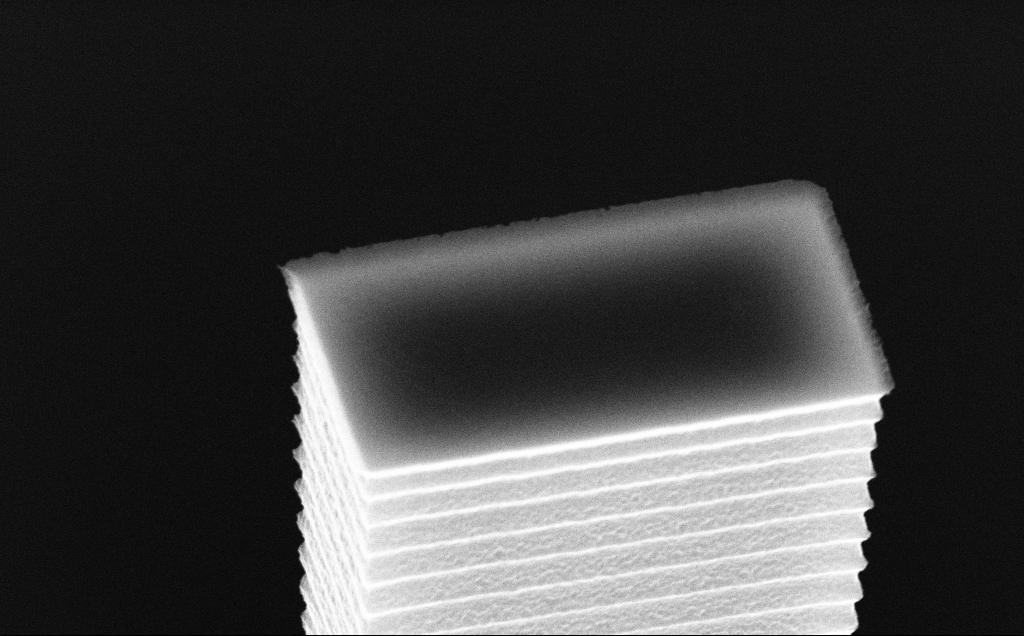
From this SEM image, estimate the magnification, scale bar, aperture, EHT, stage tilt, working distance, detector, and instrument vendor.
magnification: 42.25 K X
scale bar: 1000 nm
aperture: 30 µm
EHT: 10 kV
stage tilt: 45°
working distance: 7 mm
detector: InLens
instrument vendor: Zeiss